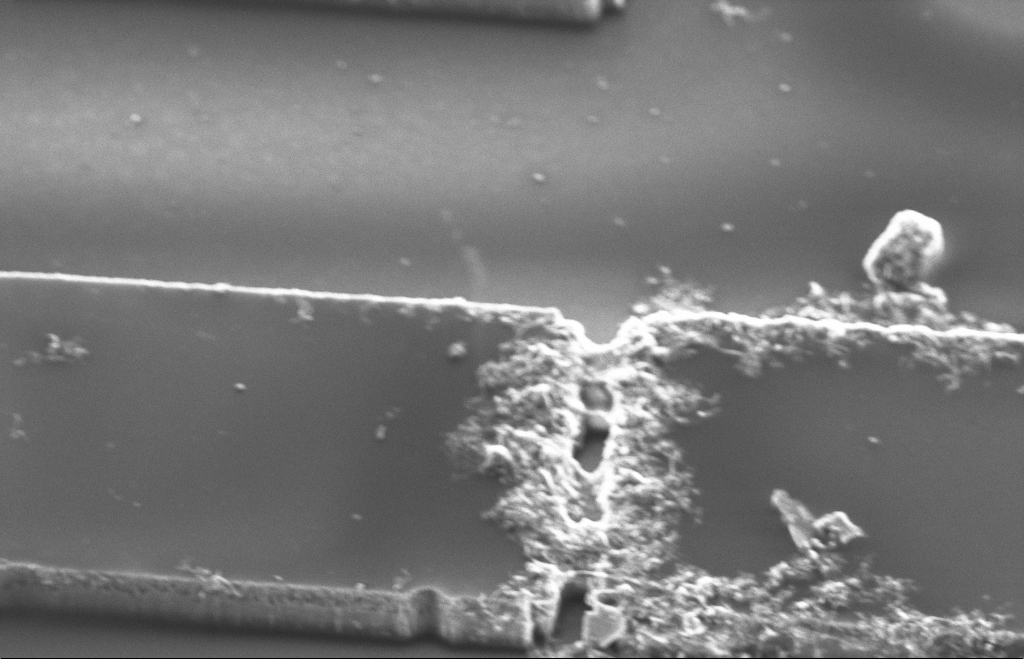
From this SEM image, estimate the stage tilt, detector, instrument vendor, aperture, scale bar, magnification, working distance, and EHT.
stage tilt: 40.8°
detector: SE2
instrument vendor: Zeiss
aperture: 20 µm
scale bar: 2000 nm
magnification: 7.38 K X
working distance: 9 mm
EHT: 5 kV